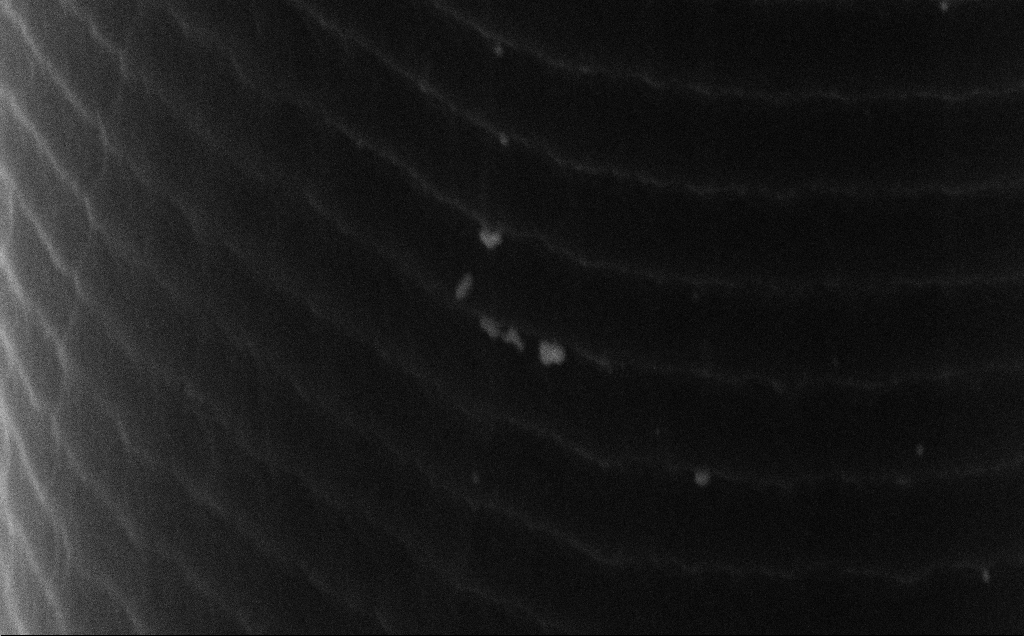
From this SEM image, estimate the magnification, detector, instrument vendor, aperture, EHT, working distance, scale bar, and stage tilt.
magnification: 95.74 K X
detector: SE2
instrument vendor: Zeiss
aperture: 30 µm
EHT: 15 kV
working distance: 10 mm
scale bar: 200 nm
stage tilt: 50°